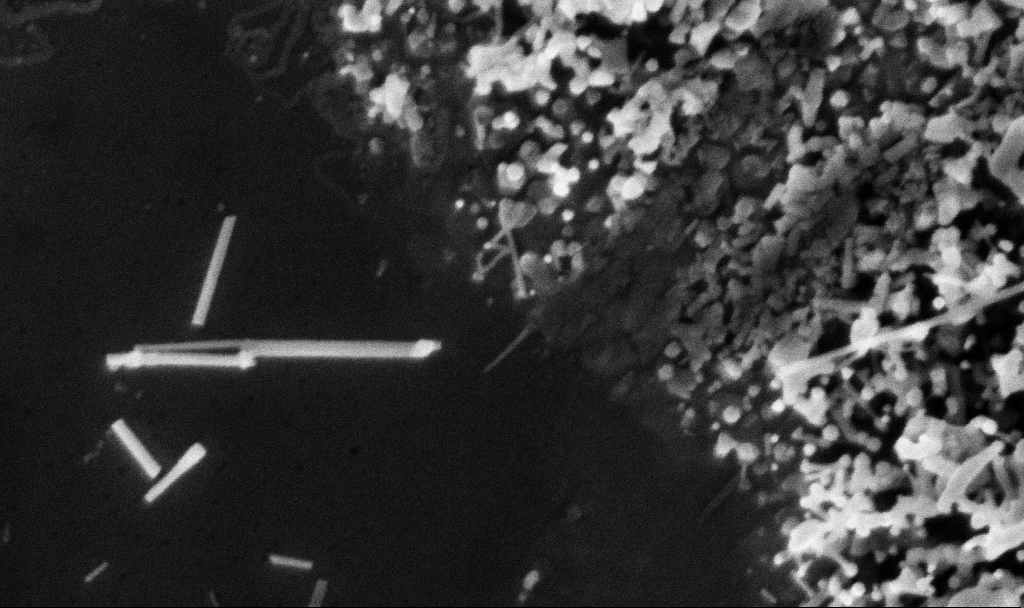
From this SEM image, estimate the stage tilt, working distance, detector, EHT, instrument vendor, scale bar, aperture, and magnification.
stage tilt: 0°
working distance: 3.1 mm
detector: InLens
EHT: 3 kV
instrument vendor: Zeiss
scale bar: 200 nm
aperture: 30 µm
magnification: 150.37 K X